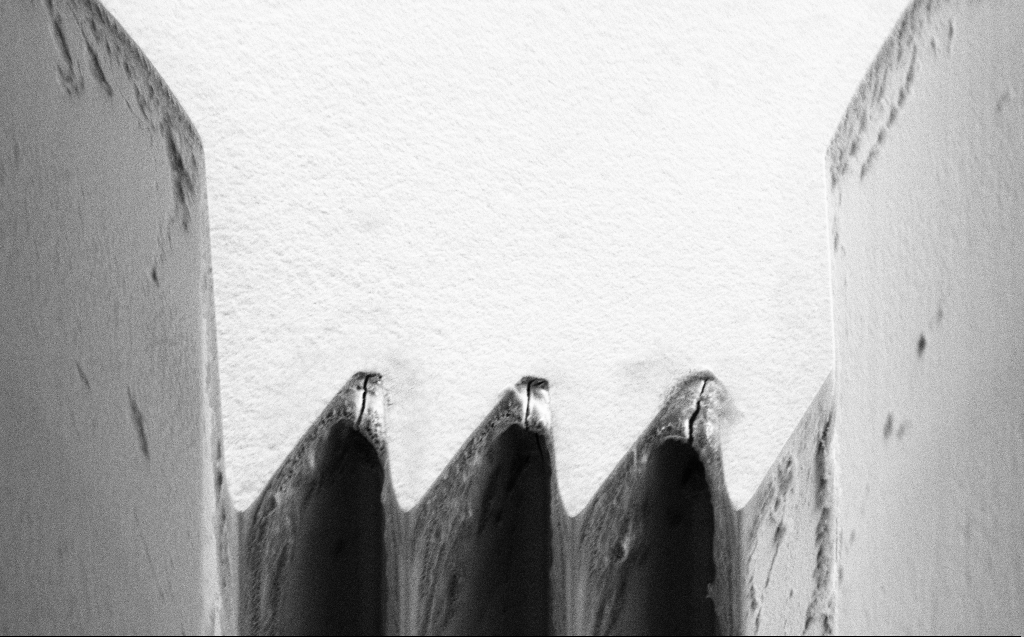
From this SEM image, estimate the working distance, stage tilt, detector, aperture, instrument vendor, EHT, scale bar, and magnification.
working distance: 7 mm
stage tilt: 45°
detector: SE2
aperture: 30 µm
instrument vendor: Zeiss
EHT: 5 kV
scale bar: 10000 nm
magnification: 5.4 K X